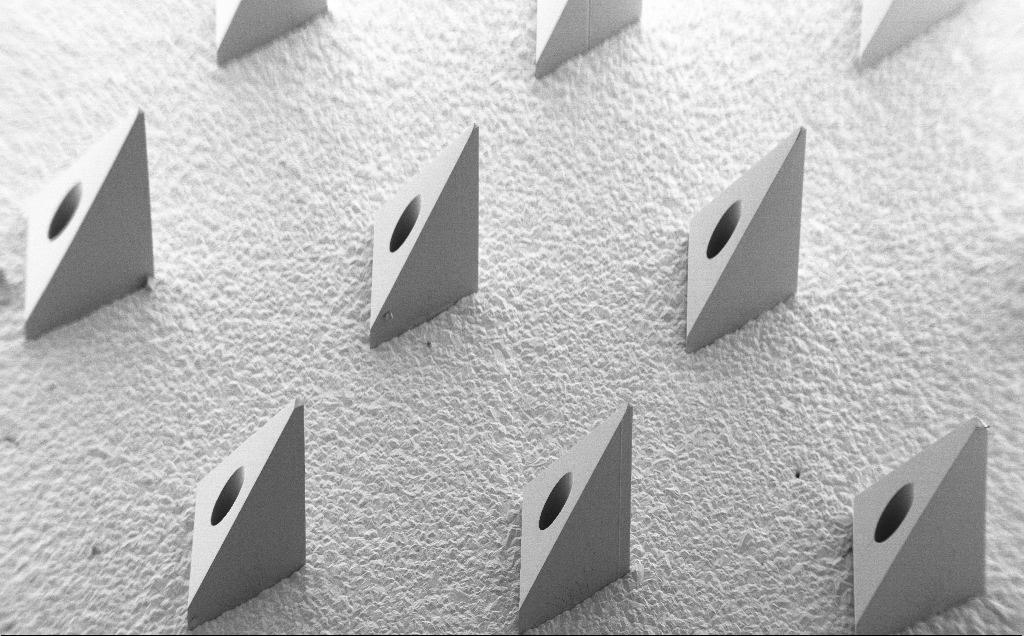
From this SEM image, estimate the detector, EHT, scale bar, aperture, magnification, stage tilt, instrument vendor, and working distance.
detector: SE2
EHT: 5 kV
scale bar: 200000 nm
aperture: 30 µm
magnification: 0.076 K X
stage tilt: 40°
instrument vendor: Zeiss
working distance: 9 mm